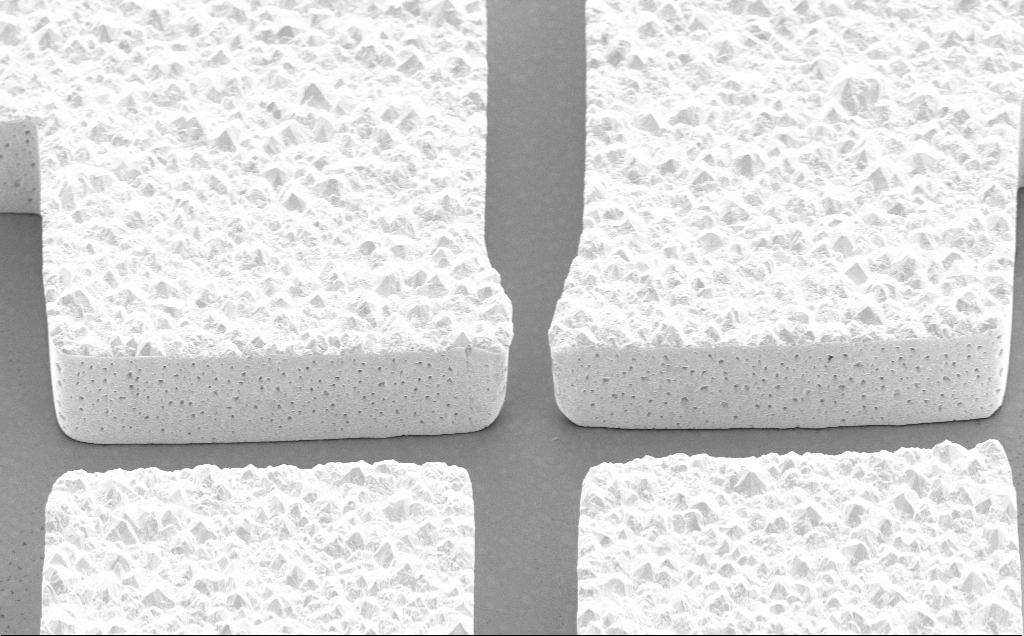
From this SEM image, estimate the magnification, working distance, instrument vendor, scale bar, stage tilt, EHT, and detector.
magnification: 4.3 K X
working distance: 14 mm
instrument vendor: Zeiss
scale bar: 10000 nm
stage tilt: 45°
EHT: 5 kV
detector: SE2